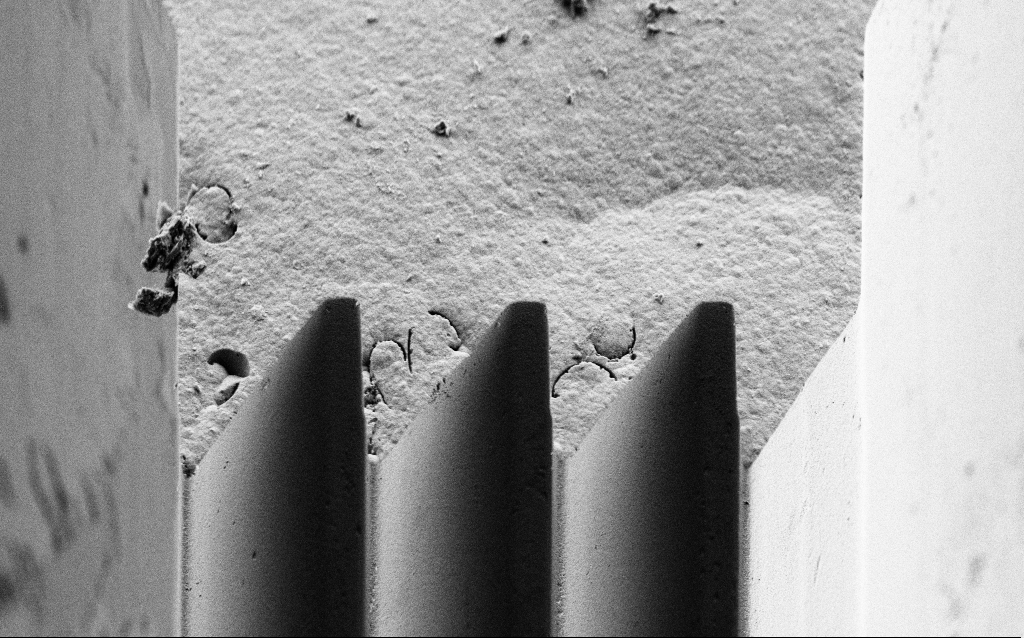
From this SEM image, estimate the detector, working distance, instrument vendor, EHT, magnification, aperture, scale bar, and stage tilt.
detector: SE2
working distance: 4 mm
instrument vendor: Zeiss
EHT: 3 kV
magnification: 6.1 K X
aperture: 30 µm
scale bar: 10000 nm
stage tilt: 45°